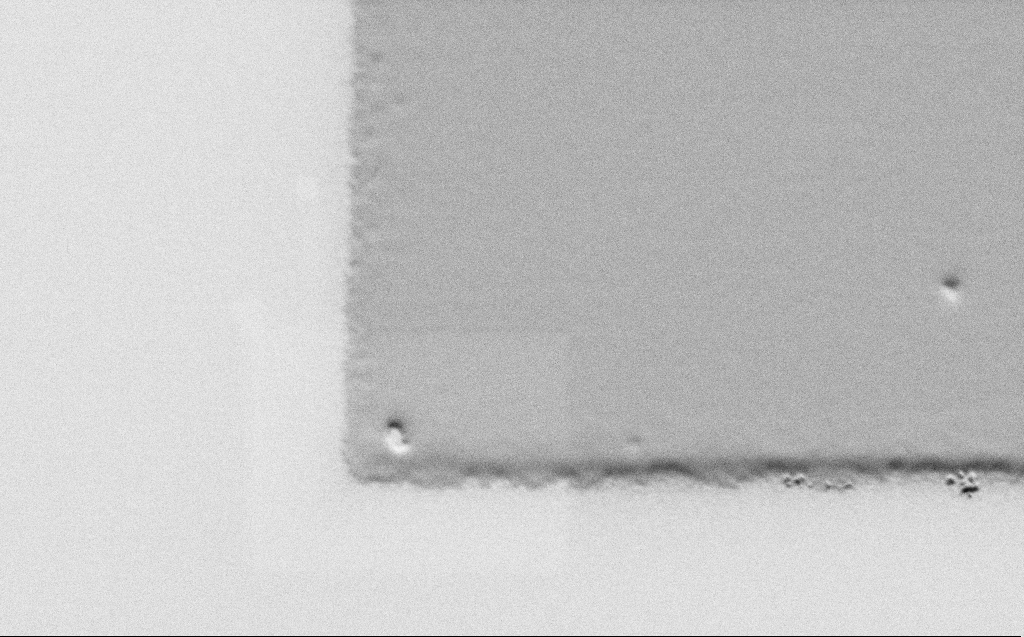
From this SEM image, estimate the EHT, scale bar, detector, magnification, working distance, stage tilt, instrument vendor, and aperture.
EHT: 1 kV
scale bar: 2000 nm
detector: SE2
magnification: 10.71 K X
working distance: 5 mm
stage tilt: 45°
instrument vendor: Zeiss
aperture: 30 µm